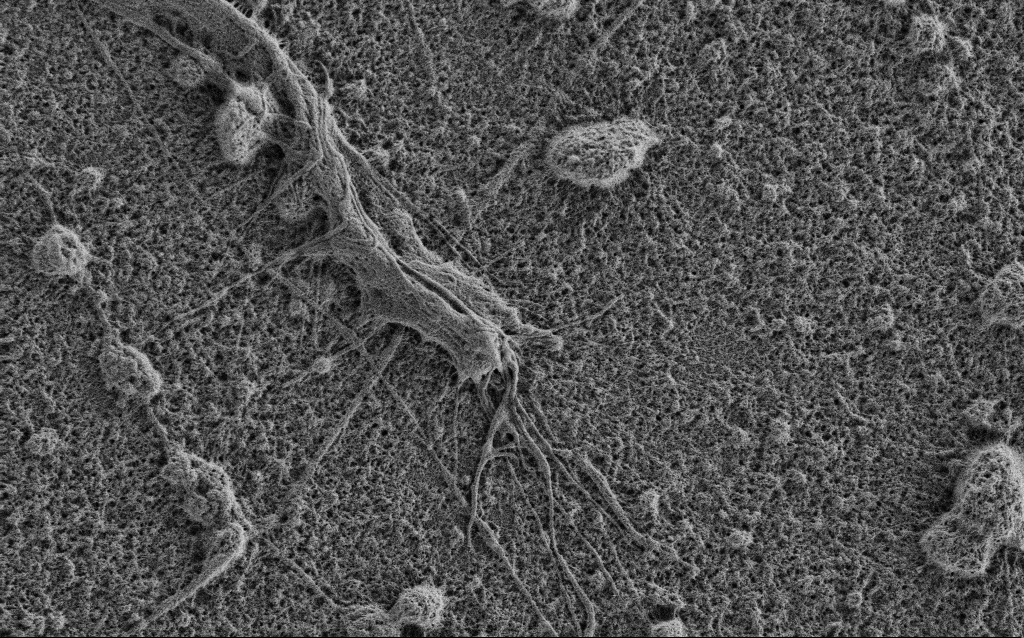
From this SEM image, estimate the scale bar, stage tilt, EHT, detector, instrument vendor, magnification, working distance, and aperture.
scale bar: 2000 nm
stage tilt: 0°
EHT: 0.9 kV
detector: SE2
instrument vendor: Zeiss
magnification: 10 K X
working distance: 3.4 mm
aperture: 30 µm